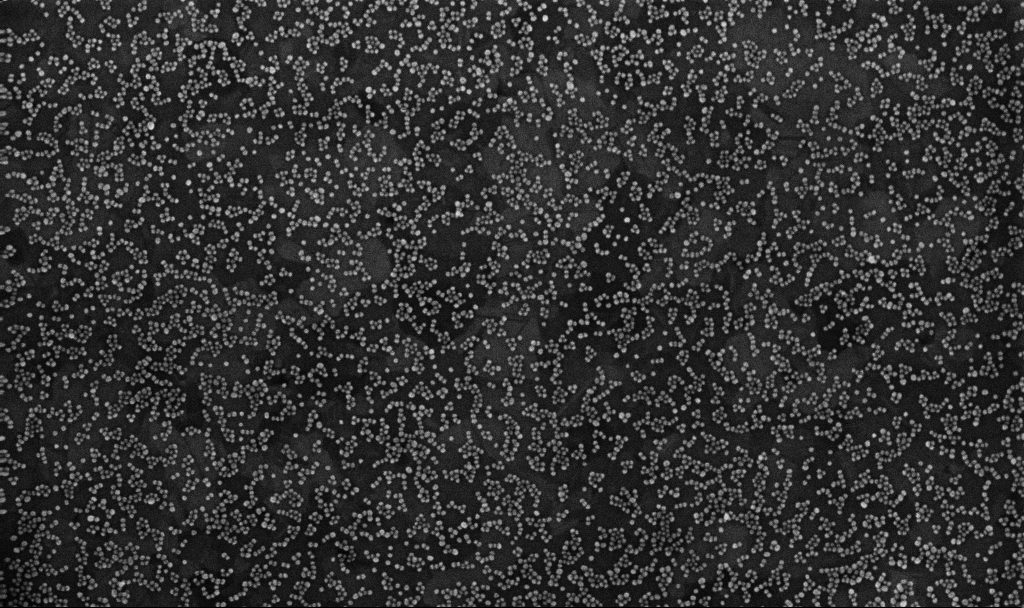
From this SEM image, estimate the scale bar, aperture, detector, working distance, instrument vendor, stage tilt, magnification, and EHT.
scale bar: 200 nm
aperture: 30 µm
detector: InLens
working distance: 3.3 mm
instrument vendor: Zeiss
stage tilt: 0°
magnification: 100 K X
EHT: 10 kV